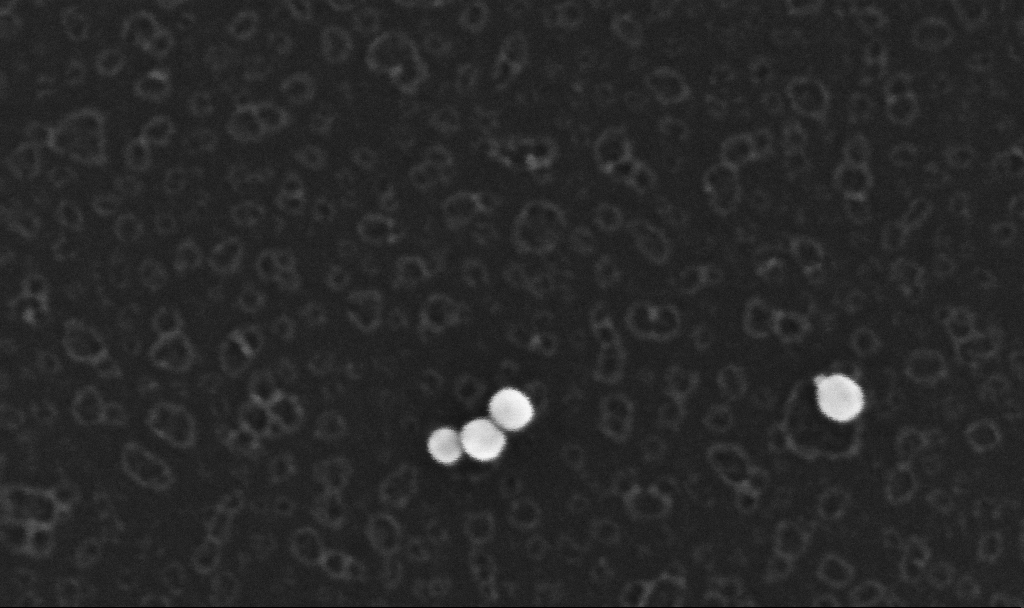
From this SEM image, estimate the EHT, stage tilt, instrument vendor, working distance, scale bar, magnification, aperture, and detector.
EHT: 10 kV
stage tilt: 0°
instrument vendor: Zeiss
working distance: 3.4 mm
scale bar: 100 nm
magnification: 500 K X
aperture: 30 µm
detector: InLens